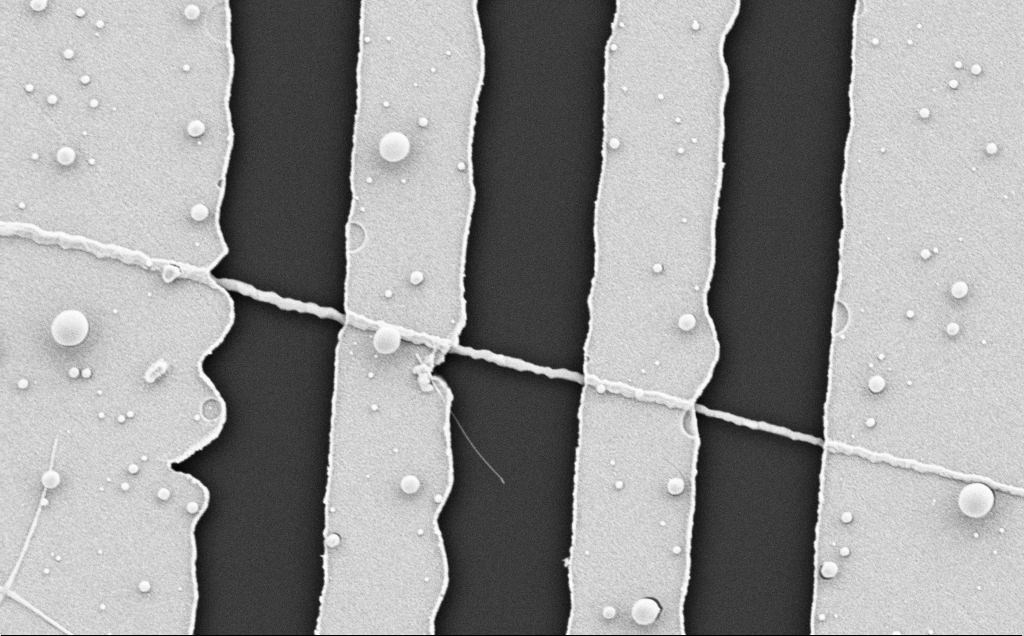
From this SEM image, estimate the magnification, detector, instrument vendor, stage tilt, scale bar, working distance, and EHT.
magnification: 22.81 K X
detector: SE2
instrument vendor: Zeiss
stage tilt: -0.7°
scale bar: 2000 nm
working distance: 8 mm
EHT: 5 kV